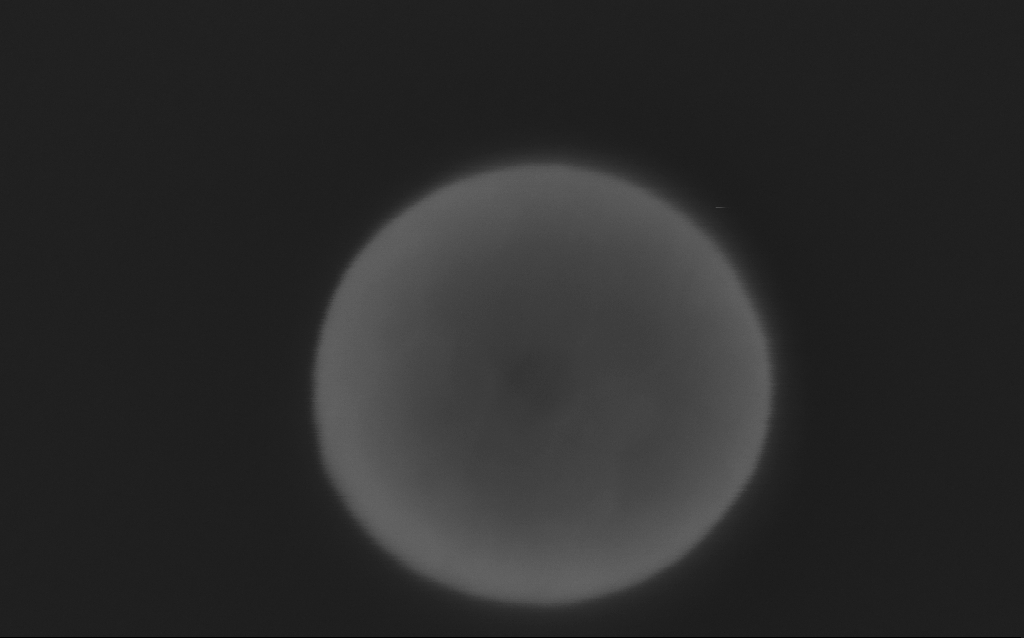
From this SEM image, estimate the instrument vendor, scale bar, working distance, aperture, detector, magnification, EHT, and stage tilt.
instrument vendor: Zeiss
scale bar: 100 nm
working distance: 3 mm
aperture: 30 µm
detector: InLens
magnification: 640.64 K X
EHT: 5 kV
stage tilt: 0°